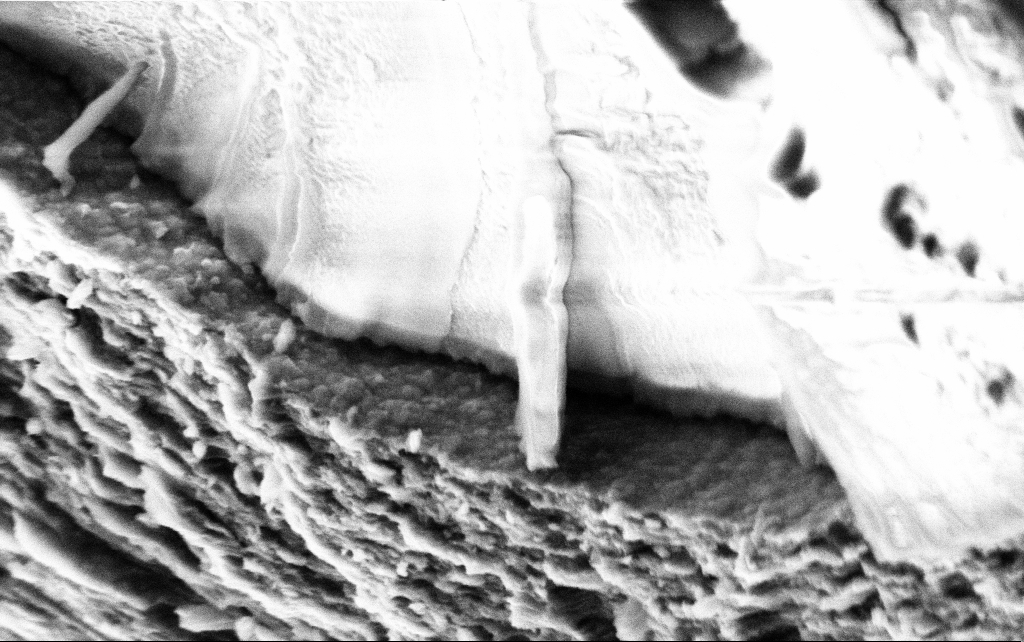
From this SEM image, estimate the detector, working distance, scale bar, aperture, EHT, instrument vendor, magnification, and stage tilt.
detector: SE2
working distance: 7.8 mm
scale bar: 1000 nm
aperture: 30 µm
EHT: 3 kV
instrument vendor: Zeiss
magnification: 50 K X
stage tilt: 45°